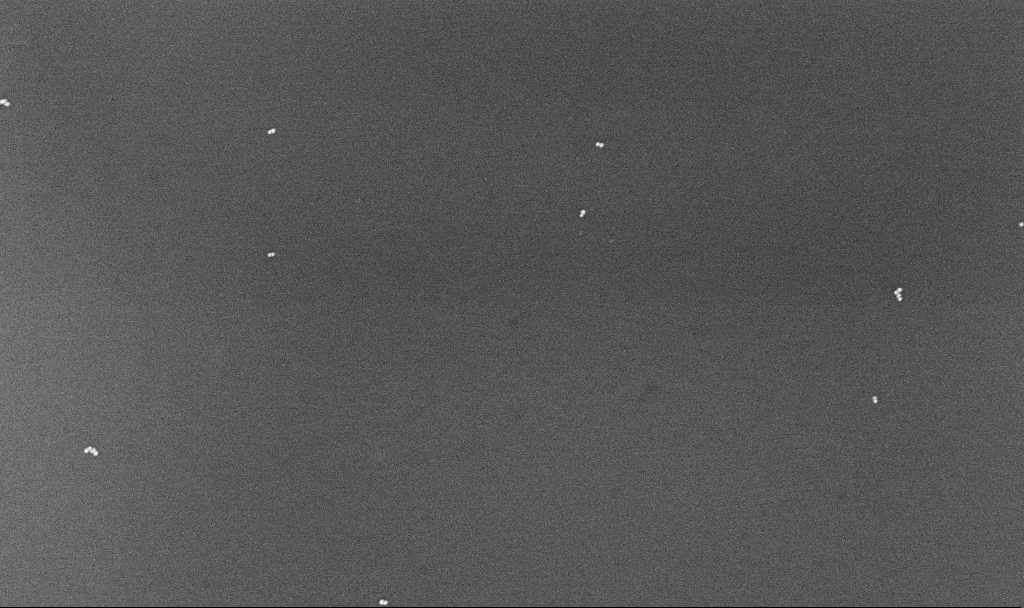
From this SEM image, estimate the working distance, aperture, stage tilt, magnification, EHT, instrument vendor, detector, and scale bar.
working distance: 4.3 mm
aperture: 30 µm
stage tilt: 0°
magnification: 86.15 K X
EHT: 10 kV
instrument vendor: Zeiss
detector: InLens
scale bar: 200 nm